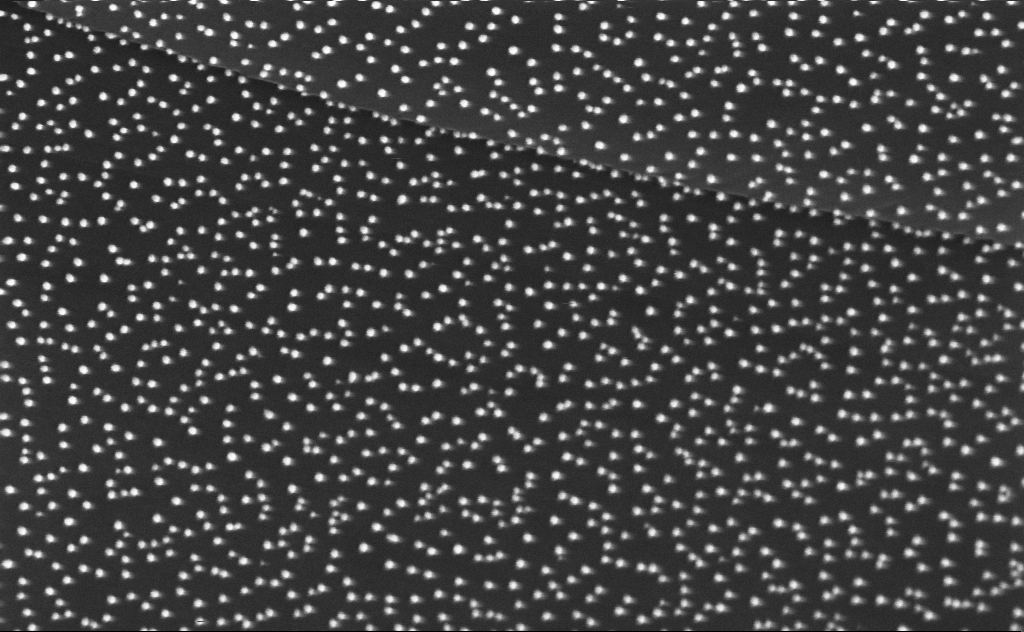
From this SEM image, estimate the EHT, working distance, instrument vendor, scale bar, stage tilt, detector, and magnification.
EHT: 3 kV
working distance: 5 mm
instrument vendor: Zeiss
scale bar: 100 nm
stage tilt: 0°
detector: InLens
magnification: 150 K X